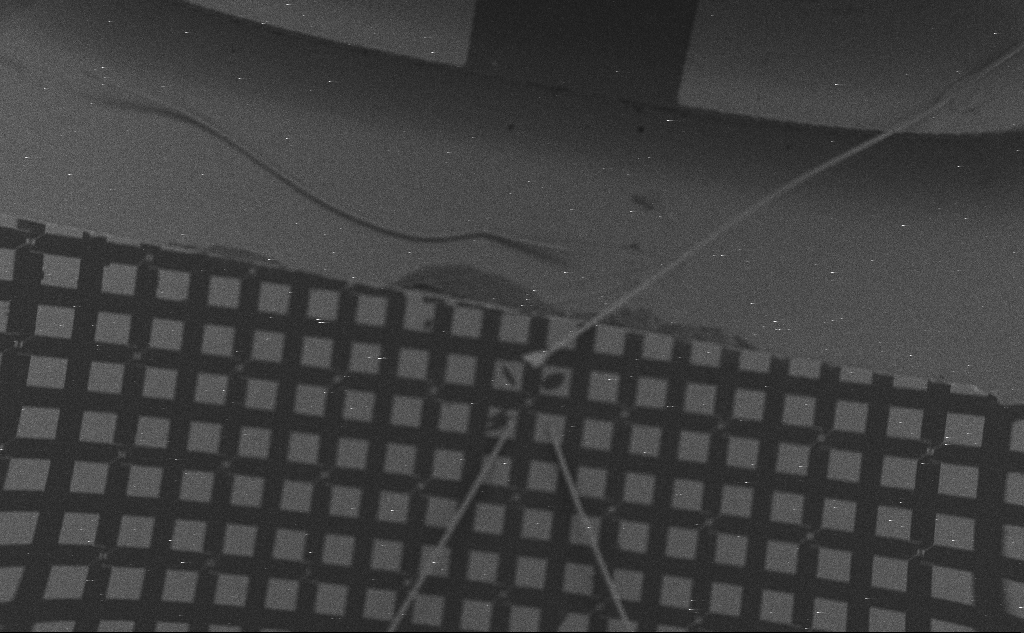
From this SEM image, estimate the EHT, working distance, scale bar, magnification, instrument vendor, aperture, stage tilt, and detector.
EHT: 5 kV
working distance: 9 mm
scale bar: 1e+06 nm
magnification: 0.067 K X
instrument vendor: Zeiss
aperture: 30 µm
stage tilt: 0°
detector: SE2